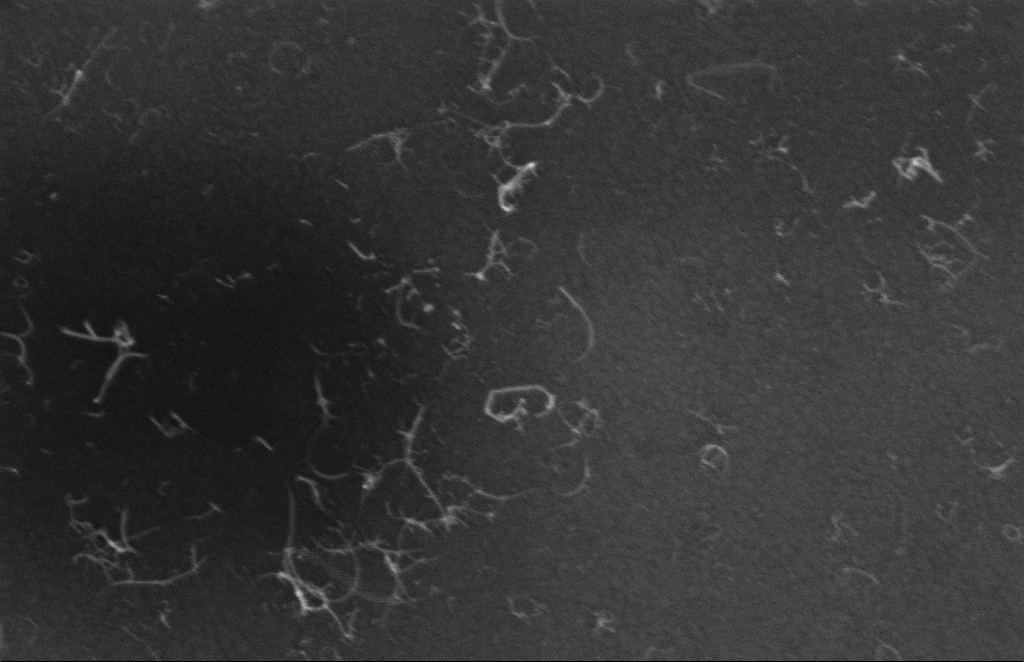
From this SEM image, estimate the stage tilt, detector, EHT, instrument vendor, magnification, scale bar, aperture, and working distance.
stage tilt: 0°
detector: InLens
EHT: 5 kV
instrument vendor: Zeiss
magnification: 191.2 K X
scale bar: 100 nm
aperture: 20 µm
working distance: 8 mm